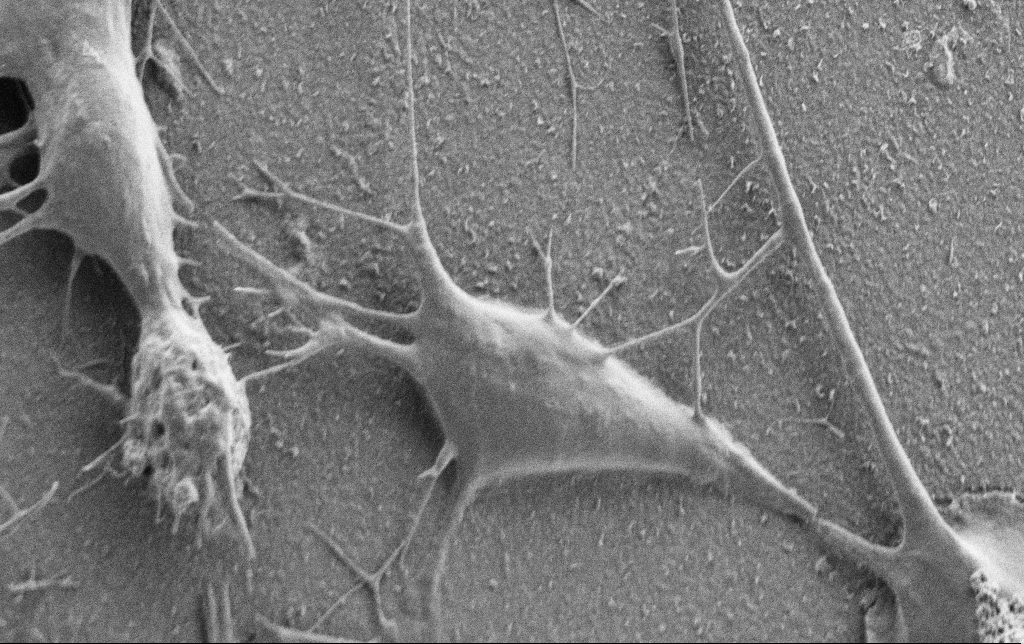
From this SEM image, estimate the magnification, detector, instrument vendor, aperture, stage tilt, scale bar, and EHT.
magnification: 10 K X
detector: SE2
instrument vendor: Zeiss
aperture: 30 µm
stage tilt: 0°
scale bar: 2000 nm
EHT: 0.9 kV